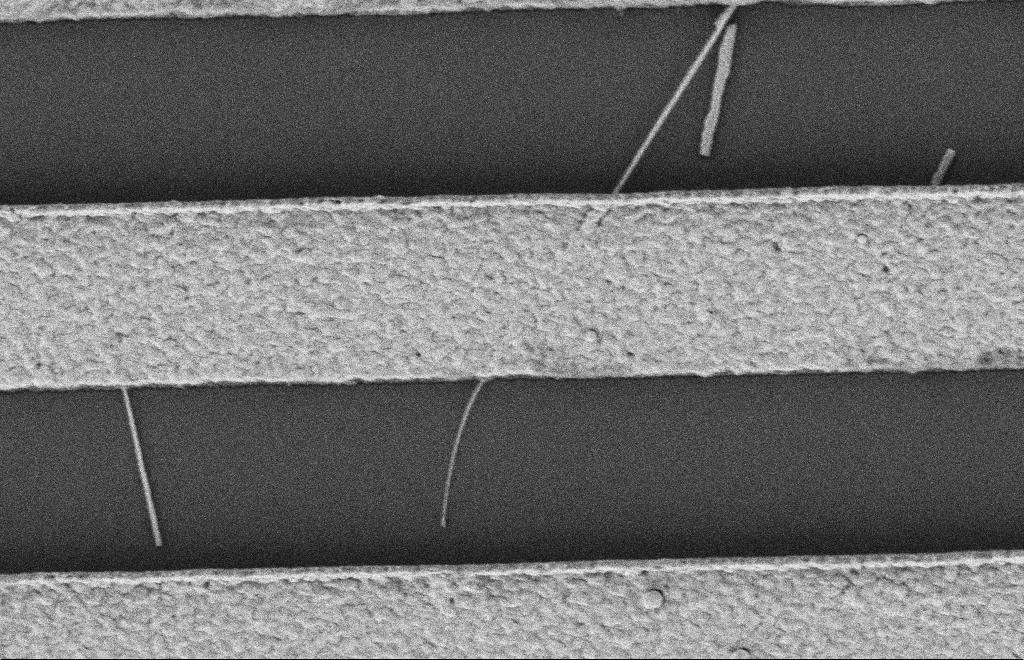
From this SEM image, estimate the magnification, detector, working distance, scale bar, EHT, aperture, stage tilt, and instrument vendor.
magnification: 33.69 K X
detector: SE2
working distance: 12 mm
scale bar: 1000 nm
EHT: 2 kV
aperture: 20 µm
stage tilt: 0°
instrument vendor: Zeiss